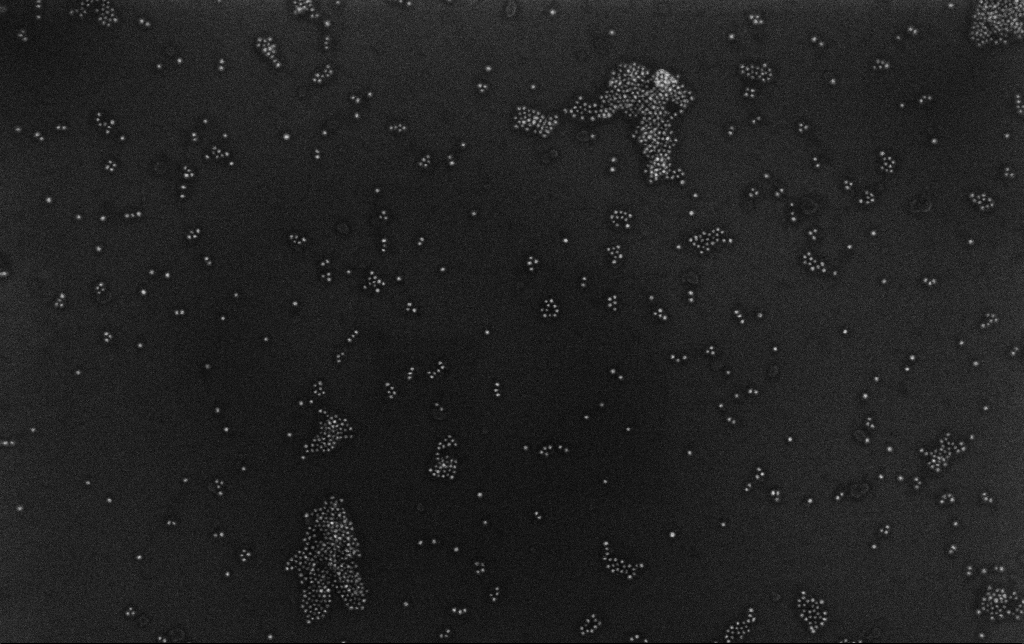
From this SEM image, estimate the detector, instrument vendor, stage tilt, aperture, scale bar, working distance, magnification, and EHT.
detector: InLens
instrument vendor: Zeiss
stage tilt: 0°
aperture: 30 µm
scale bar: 200 nm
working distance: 3.4 mm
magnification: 100 K X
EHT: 10 kV